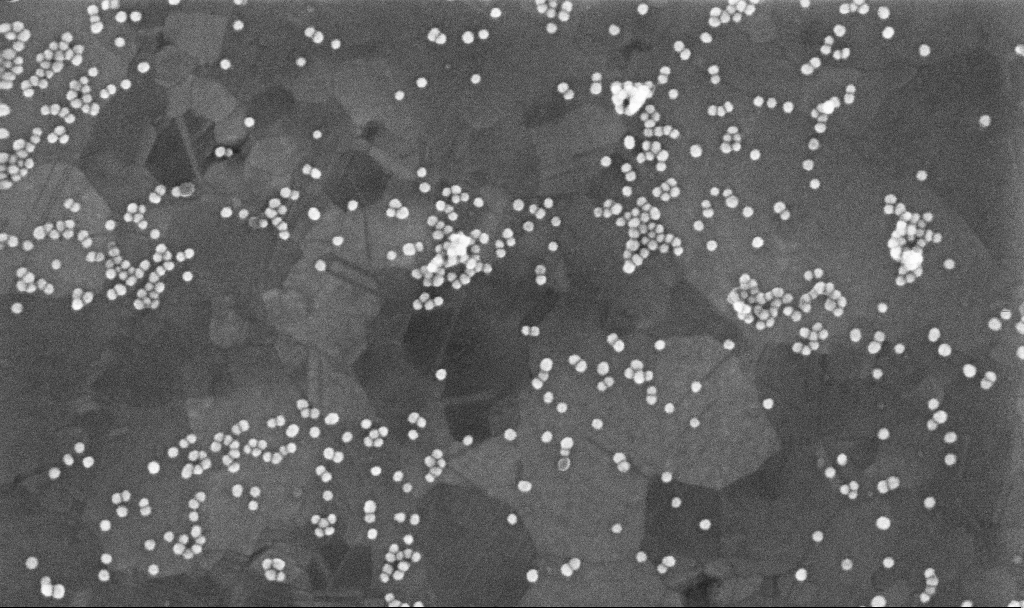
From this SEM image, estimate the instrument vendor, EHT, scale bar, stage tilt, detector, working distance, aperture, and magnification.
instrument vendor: Zeiss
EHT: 10 kV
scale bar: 100 nm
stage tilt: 0°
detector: InLens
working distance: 3.7 mm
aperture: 30 µm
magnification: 200 K X